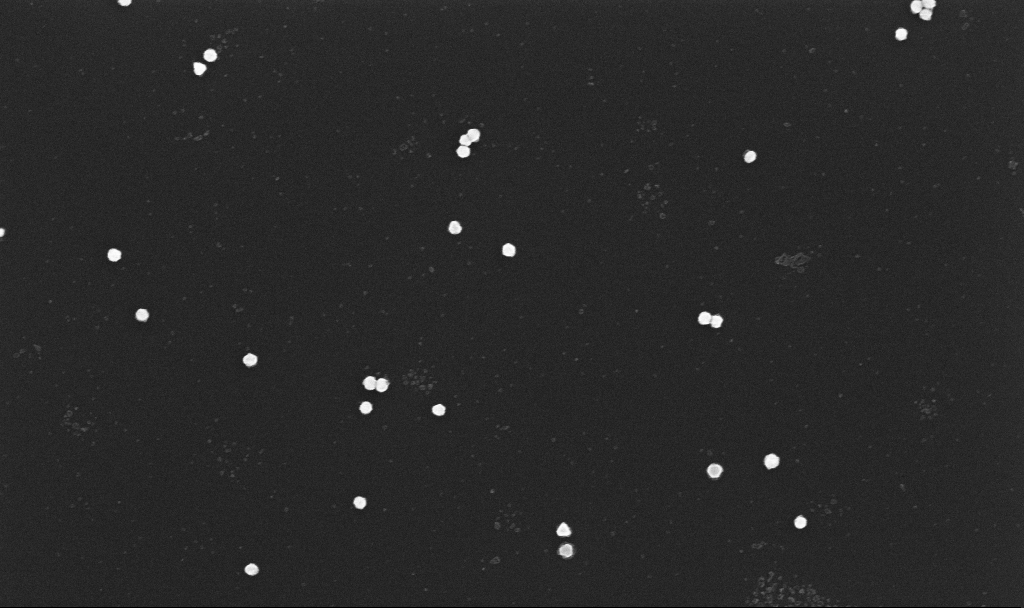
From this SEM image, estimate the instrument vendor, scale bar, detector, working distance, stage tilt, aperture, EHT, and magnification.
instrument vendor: Zeiss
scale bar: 1000 nm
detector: InLens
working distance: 3.3 mm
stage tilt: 0°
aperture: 30 µm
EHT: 10 kV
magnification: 70 K X